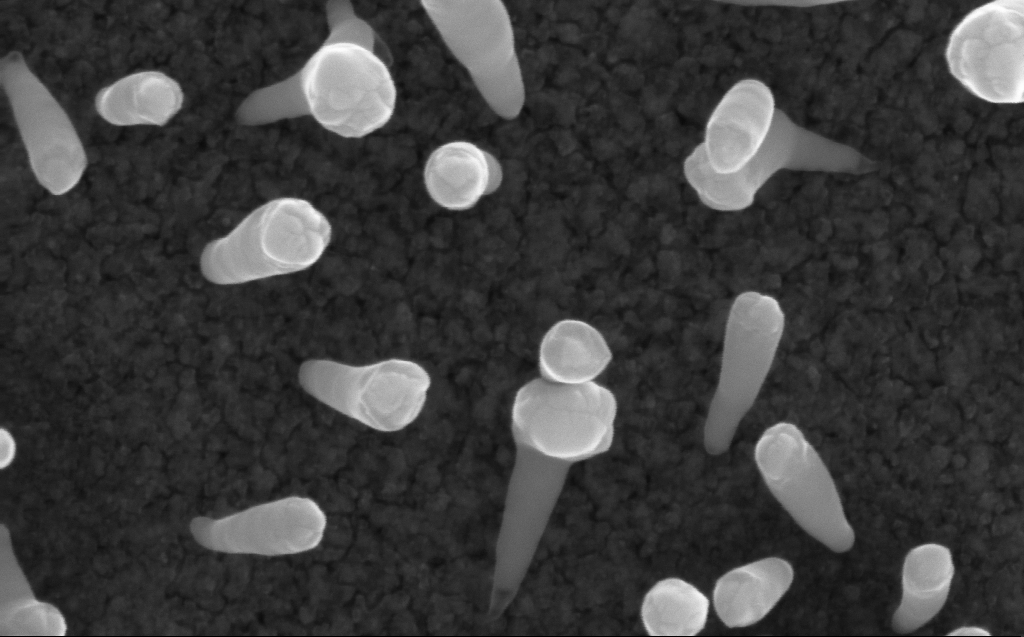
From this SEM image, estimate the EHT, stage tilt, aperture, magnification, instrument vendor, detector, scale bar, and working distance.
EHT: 10 kV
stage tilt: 0°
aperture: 30 µm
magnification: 200 K X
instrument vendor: Zeiss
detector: InLens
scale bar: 200 nm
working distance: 3 mm